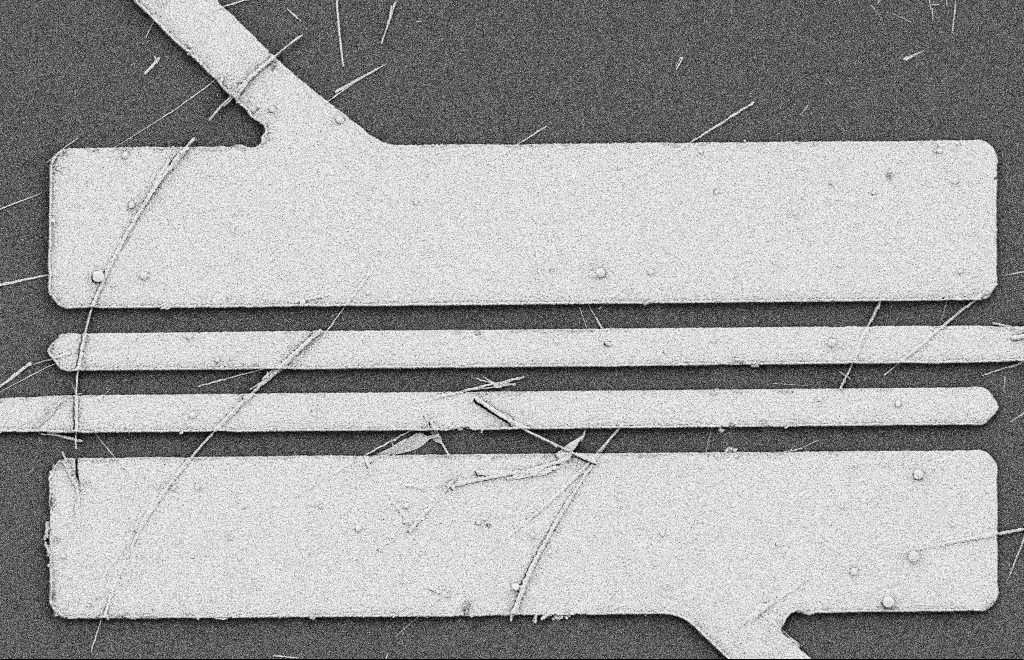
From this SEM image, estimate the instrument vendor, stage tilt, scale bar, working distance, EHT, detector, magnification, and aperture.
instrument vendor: Zeiss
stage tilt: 0°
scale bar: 2000 nm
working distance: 10 mm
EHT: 2 kV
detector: SE2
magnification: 5.69 K X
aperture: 20 µm